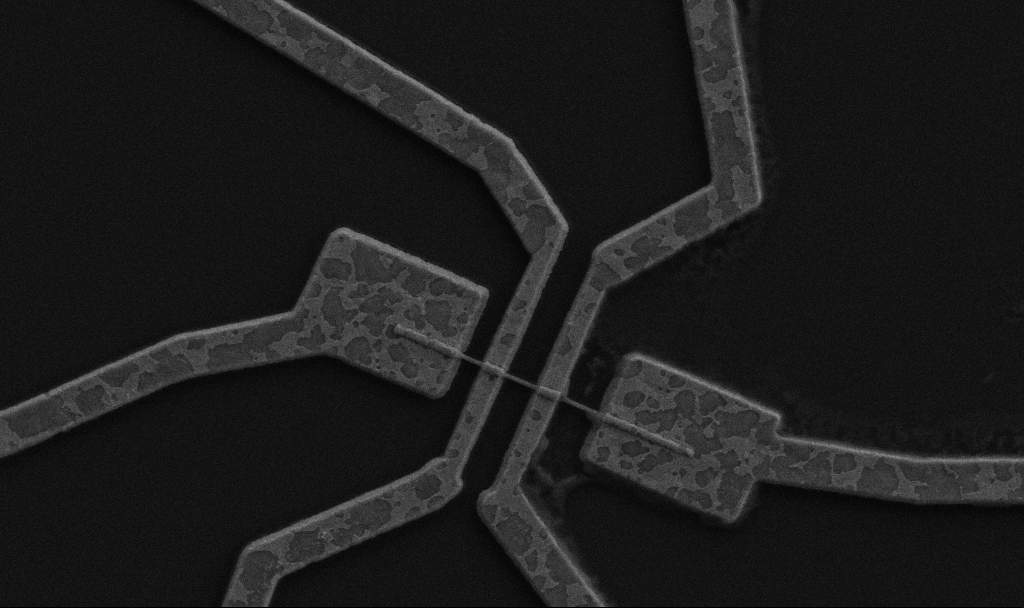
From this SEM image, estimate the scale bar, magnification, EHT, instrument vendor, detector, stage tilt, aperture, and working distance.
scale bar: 1000 nm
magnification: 20 K X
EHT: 5 kV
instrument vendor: Zeiss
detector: SE2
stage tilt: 0°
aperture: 30 µm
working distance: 8.7 mm